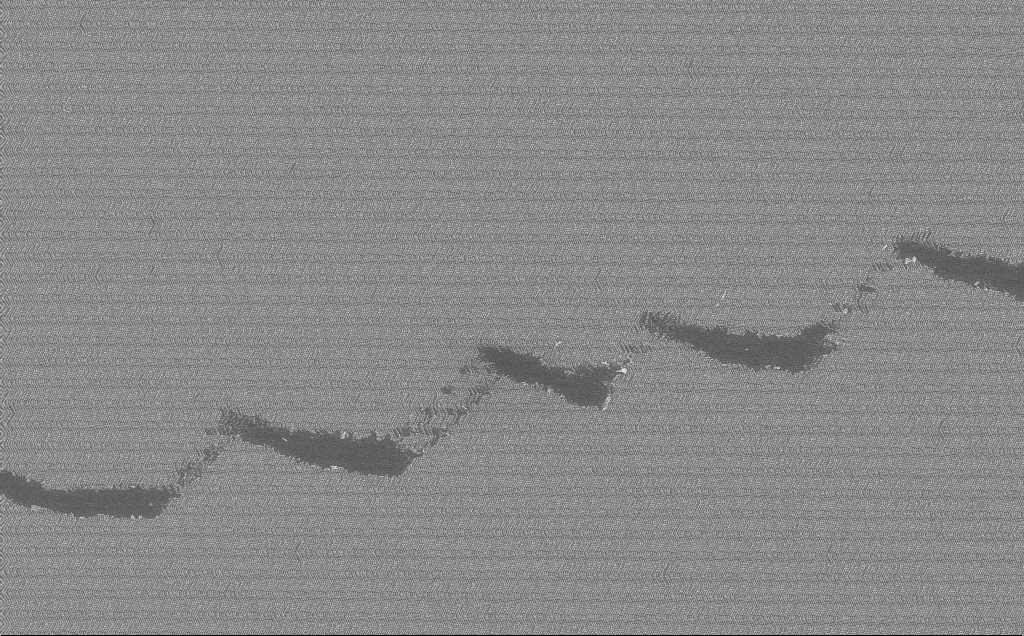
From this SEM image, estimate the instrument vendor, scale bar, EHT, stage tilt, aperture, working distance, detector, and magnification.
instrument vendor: Zeiss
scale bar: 10000 nm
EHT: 10 kV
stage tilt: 0°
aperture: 30 µm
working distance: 7 mm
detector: InLens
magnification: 3.91 K X